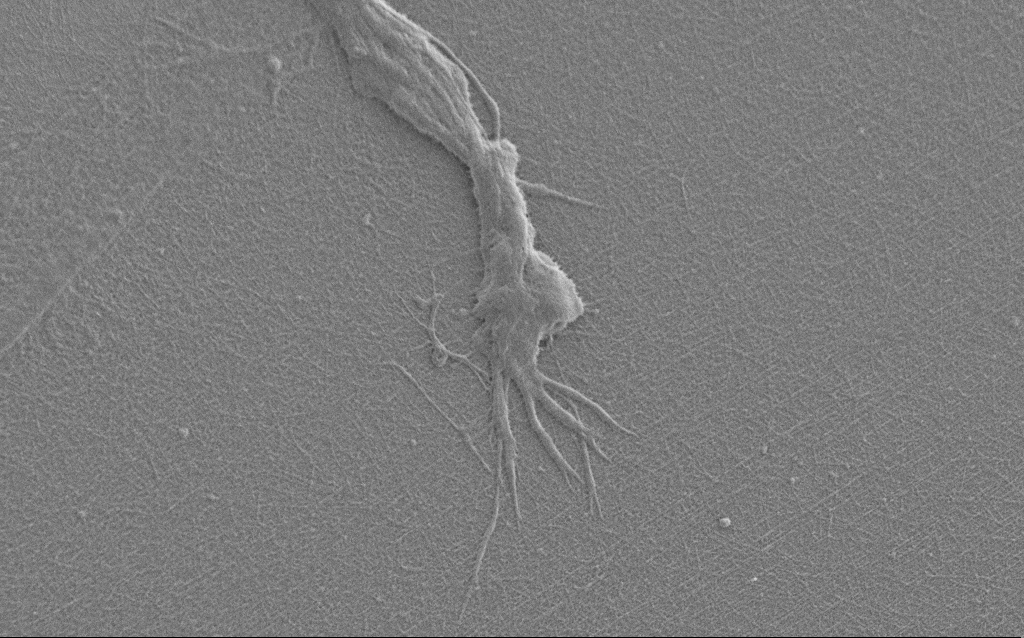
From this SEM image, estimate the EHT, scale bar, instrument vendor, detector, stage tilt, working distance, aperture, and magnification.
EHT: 1 kV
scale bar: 2000 nm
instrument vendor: Zeiss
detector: SE2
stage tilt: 0°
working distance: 6 mm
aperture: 30 µm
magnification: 7.5 K X